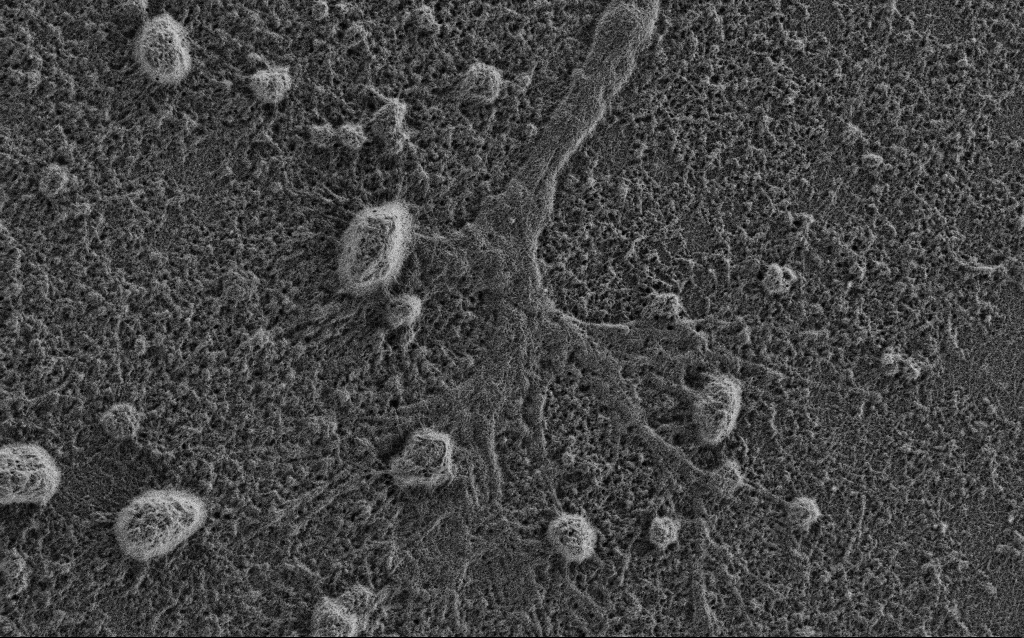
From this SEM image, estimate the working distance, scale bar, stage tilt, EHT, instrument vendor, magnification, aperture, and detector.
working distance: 3.4 mm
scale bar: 2000 nm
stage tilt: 0°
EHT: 0.9 kV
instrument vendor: Zeiss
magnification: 10 K X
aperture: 30 µm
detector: SE2